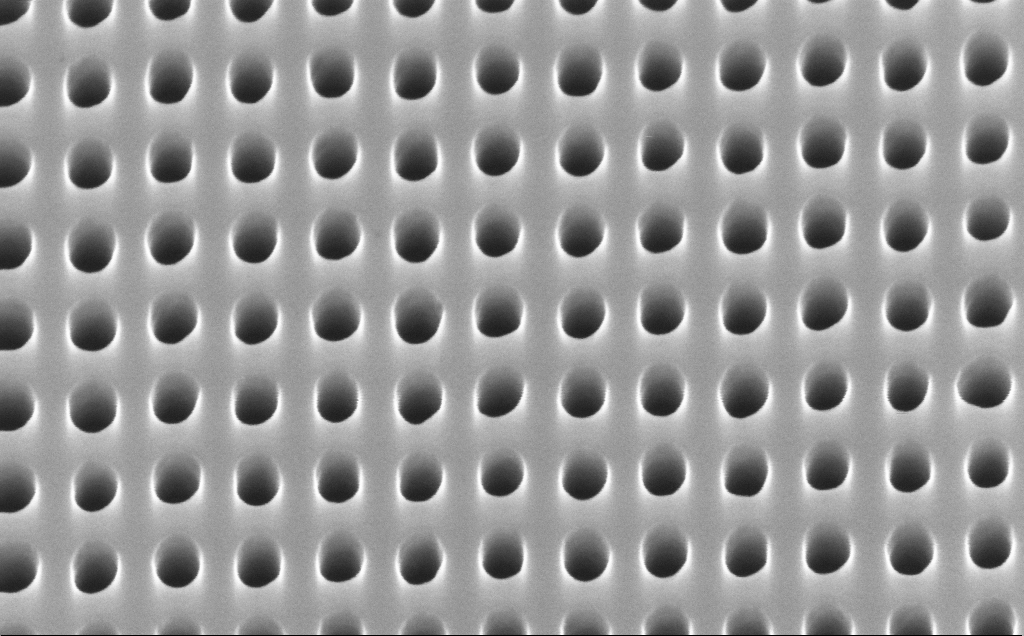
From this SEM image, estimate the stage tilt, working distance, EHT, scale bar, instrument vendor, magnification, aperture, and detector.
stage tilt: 30°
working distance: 4 mm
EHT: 10 kV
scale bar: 100 nm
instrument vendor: Zeiss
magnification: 200 K X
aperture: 30 µm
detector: InLens